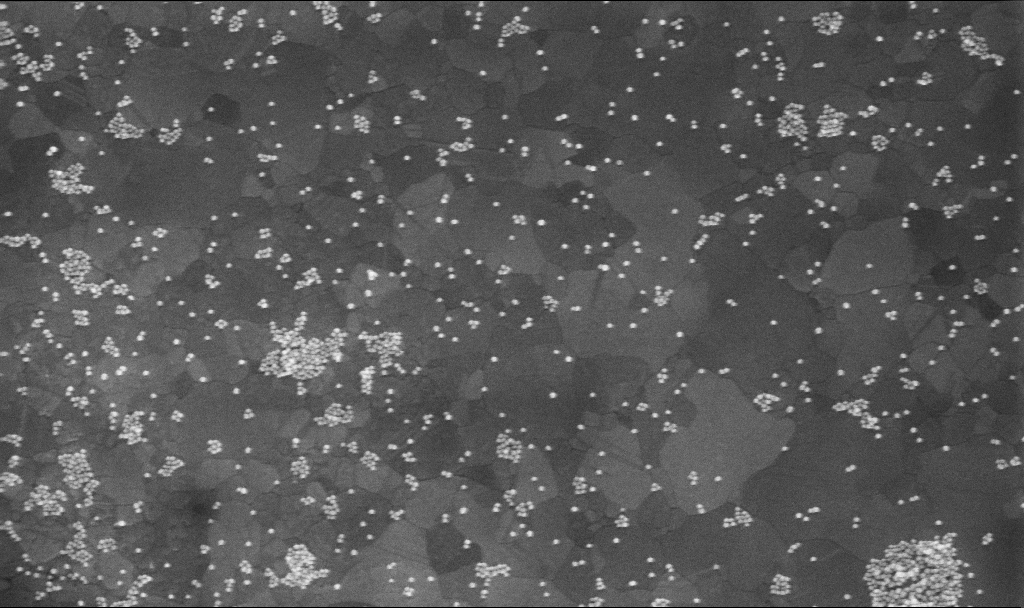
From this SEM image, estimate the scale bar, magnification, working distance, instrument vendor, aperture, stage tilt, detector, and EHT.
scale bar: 200 nm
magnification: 100 K X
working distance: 3.8 mm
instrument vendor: Zeiss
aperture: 30 µm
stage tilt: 0°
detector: InLens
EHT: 10 kV